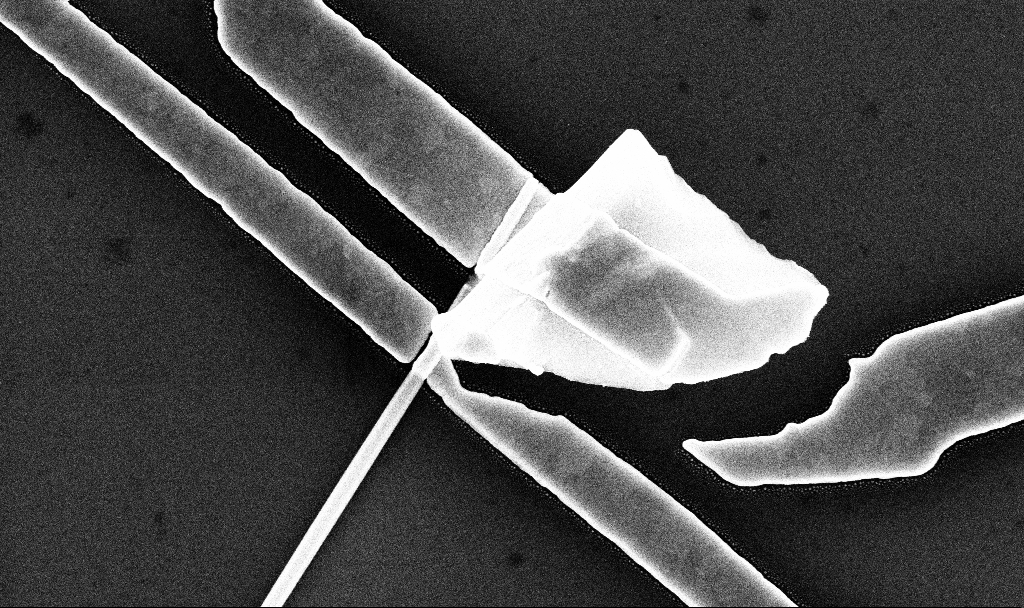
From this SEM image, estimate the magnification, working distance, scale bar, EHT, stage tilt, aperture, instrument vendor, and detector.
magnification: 46.63 K X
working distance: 7.7 mm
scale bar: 1000 nm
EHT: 10 kV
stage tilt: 0°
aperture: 30 µm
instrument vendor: Zeiss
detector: InLens